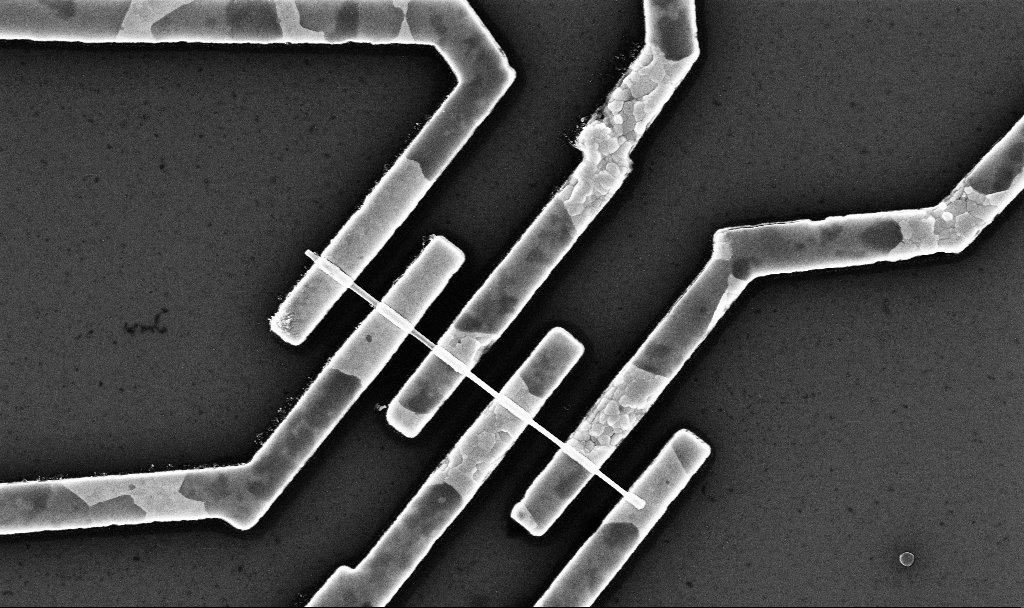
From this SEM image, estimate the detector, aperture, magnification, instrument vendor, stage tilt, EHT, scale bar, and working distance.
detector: InLens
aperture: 30 µm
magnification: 25.37 K X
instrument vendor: Zeiss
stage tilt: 0°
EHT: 10 kV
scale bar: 2000 nm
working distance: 6.8 mm